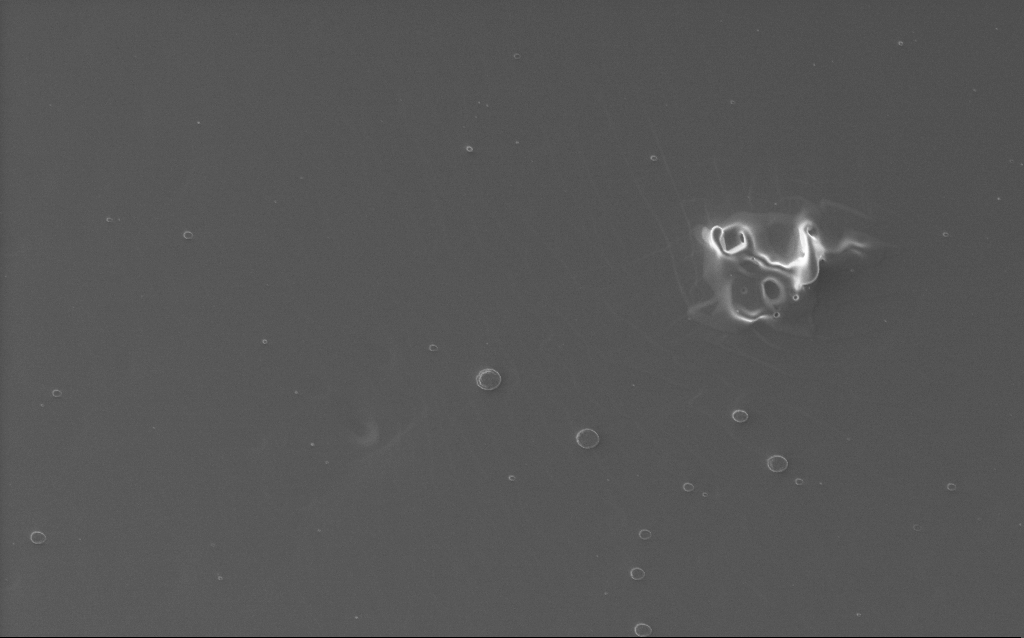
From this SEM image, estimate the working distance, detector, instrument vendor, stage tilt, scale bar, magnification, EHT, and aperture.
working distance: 2 mm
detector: InLens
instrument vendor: Zeiss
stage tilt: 0°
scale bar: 10000 nm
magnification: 4.63 K X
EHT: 5 kV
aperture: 30 µm